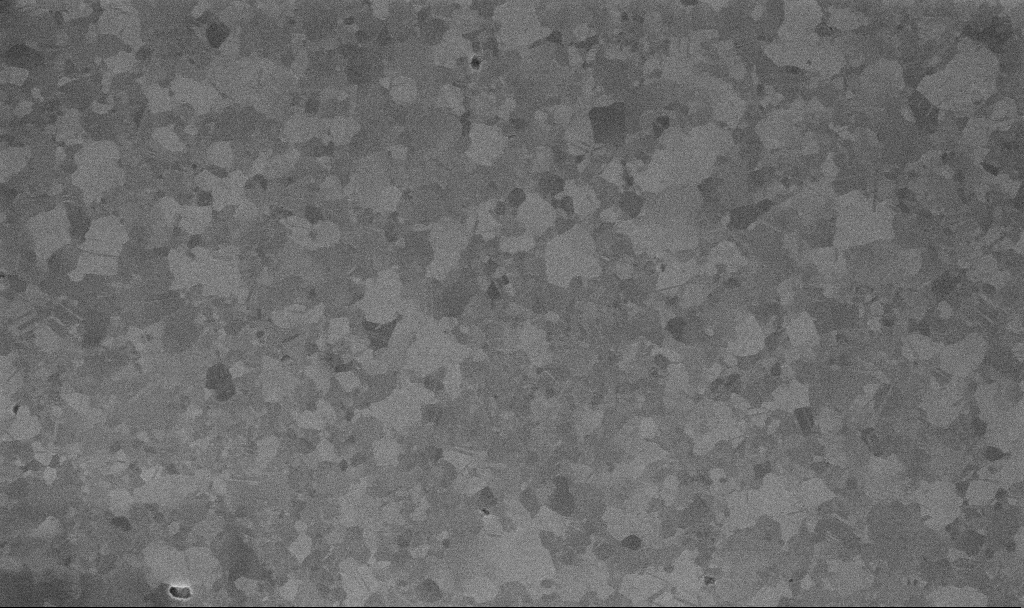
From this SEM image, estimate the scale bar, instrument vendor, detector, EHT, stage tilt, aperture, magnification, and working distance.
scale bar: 1000 nm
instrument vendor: Zeiss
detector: InLens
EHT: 10 kV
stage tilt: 0°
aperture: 30 µm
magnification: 50 K X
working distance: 3.4 mm